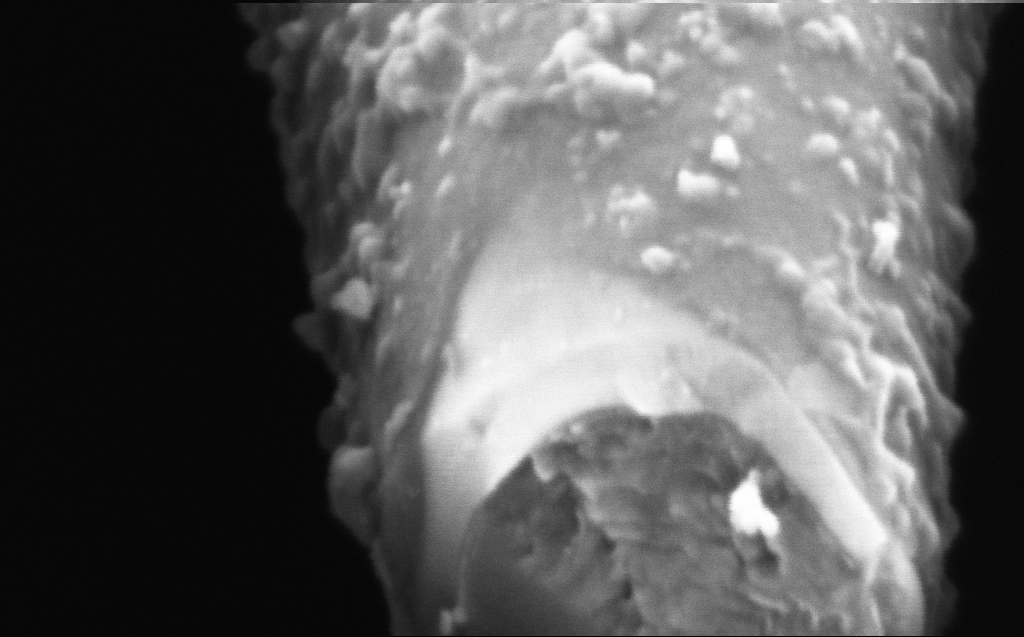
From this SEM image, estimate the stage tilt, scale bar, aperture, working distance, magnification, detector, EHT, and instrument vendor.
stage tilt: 45°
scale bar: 100 nm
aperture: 30 µm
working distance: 4 mm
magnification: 400 K X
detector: InLens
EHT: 2 kV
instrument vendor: Zeiss